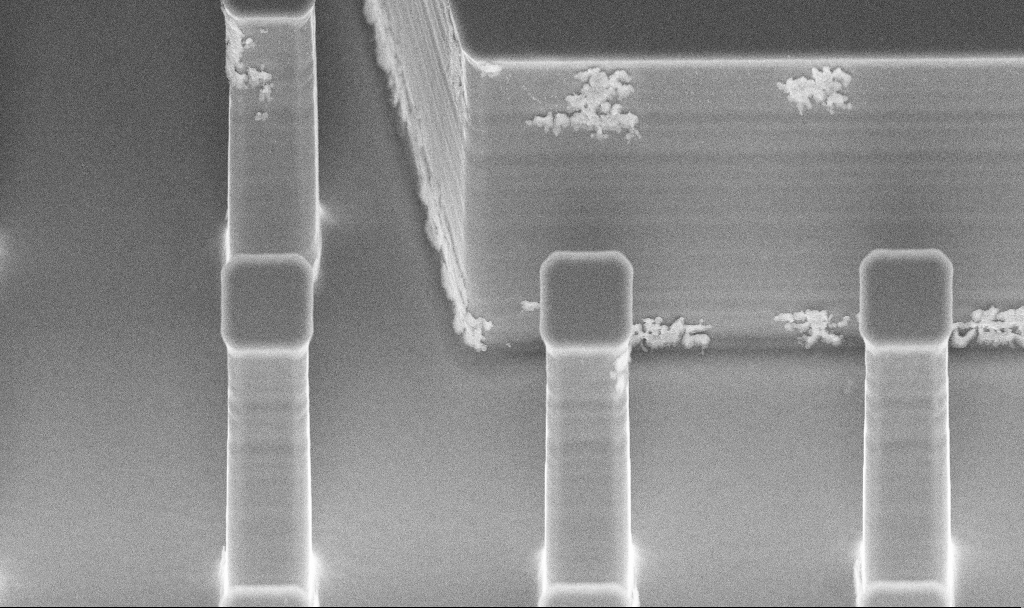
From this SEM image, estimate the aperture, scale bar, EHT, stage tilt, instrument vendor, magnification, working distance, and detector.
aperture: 30 µm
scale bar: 10000 nm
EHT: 10 kV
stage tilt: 45°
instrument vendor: Zeiss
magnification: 3.92 K X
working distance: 10 mm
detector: InLens